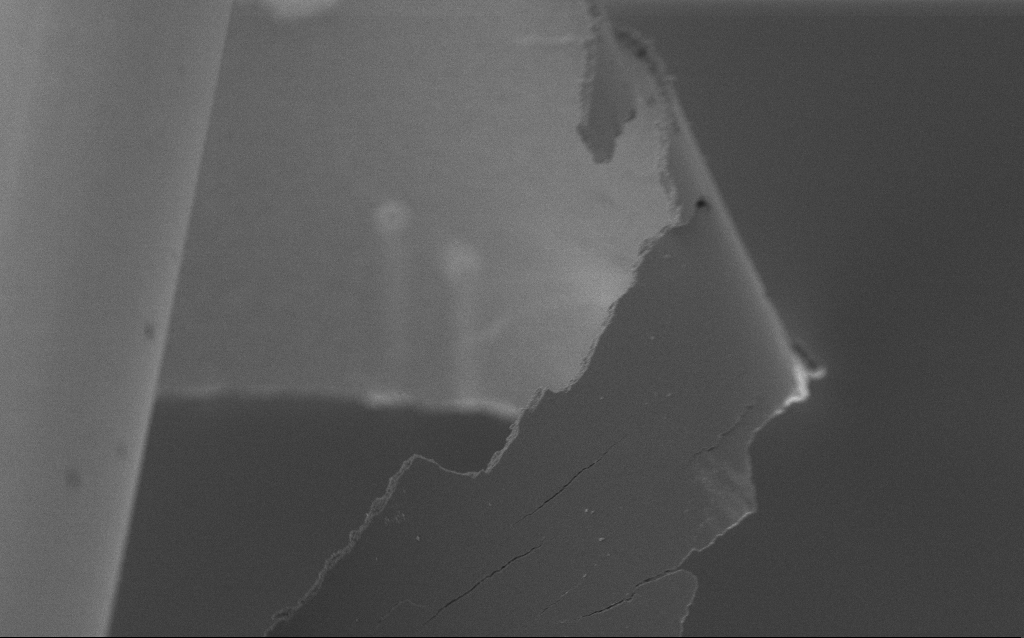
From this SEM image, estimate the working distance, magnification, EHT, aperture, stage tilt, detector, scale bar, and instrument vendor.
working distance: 5 mm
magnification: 12.55 K X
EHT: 1 kV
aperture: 30 µm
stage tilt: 45°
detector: InLens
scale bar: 2000 nm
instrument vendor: Zeiss